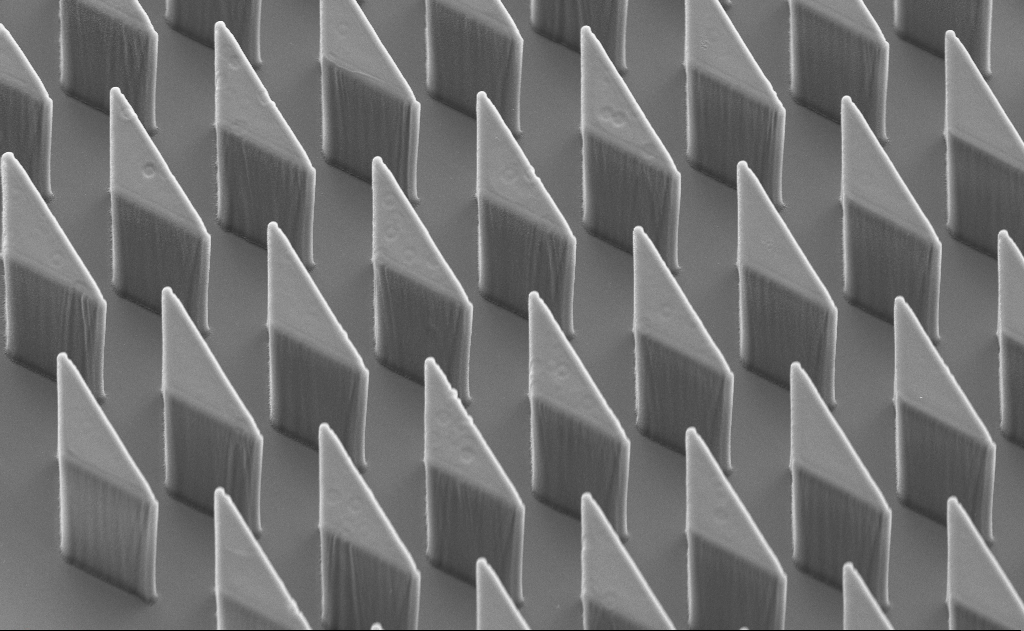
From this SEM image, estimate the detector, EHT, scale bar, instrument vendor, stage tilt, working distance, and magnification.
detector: SE2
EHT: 10 kV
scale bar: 10000 nm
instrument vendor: Zeiss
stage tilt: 45°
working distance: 10 mm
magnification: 1.89 K X